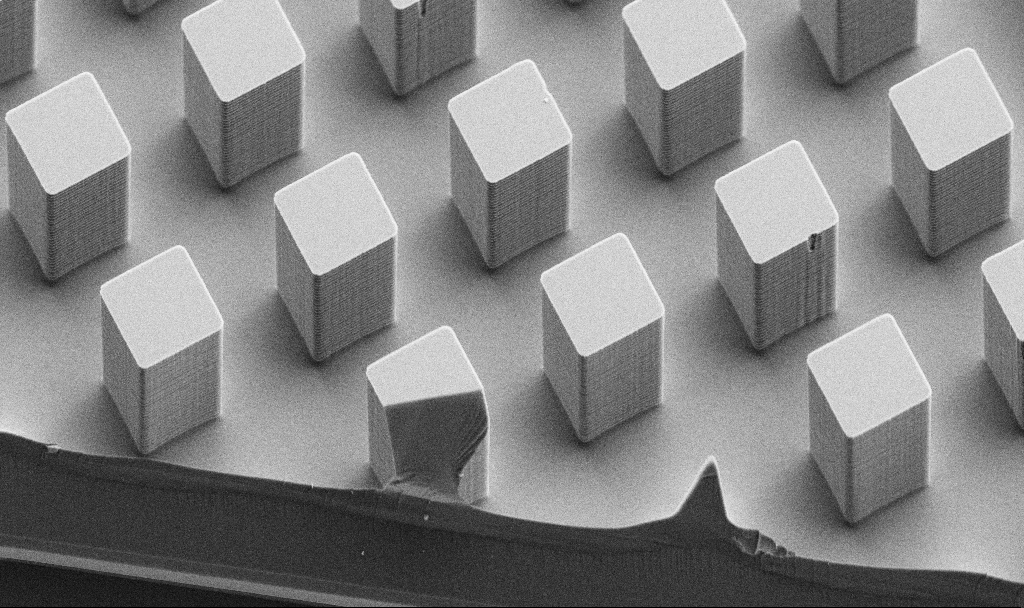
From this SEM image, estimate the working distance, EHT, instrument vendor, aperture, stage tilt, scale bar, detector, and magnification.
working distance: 6.5 mm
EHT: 5 kV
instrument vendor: Zeiss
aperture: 30 µm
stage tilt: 45°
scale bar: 10000 nm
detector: SE2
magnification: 3.63 K X